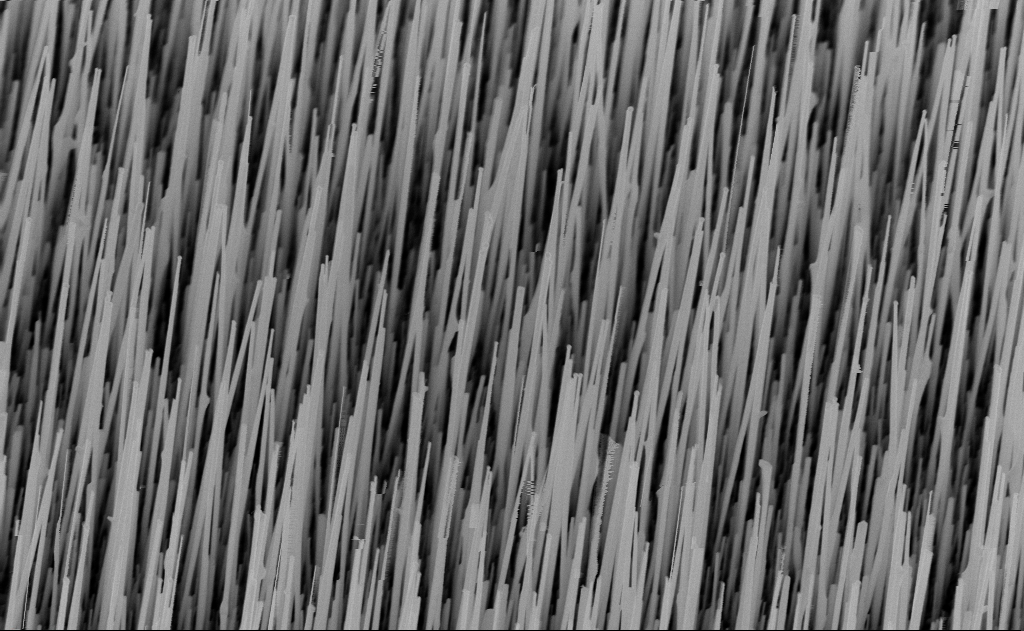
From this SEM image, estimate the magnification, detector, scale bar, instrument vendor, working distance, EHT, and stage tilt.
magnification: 20 K X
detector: InLens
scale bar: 2000 nm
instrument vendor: Zeiss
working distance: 9 mm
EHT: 10 kV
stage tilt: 30°